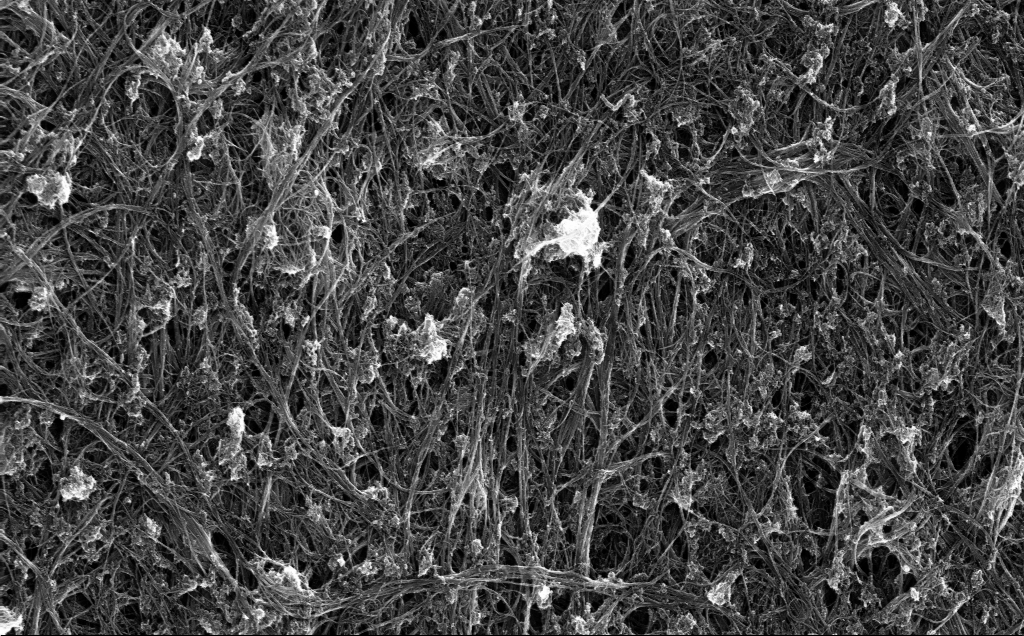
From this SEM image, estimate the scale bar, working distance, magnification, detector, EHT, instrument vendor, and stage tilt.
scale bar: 2000 nm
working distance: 4 mm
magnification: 33.79 K X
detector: InLens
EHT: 5 kV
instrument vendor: Zeiss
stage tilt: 0°